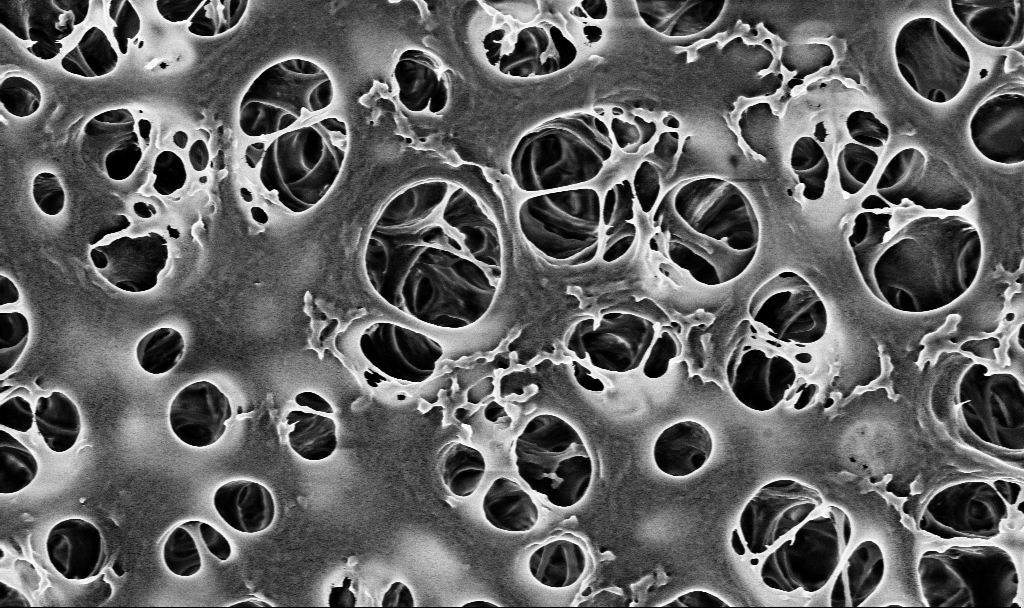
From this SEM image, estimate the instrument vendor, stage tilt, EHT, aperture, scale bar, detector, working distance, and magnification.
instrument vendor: Zeiss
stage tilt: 0°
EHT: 2 kV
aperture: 30 µm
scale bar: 2000 nm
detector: InLens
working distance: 3.7 mm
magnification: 25 K X